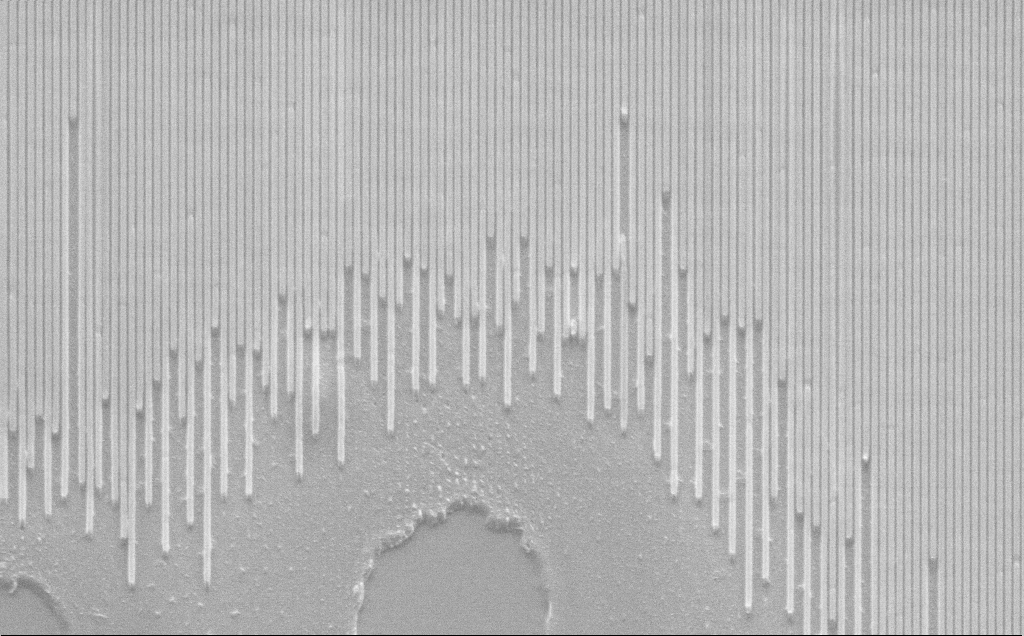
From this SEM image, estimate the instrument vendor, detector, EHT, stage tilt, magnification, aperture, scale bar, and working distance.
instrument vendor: Zeiss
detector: SE2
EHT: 10 kV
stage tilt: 45°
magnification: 17.2 K X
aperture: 30 µm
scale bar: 2000 nm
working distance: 8 mm